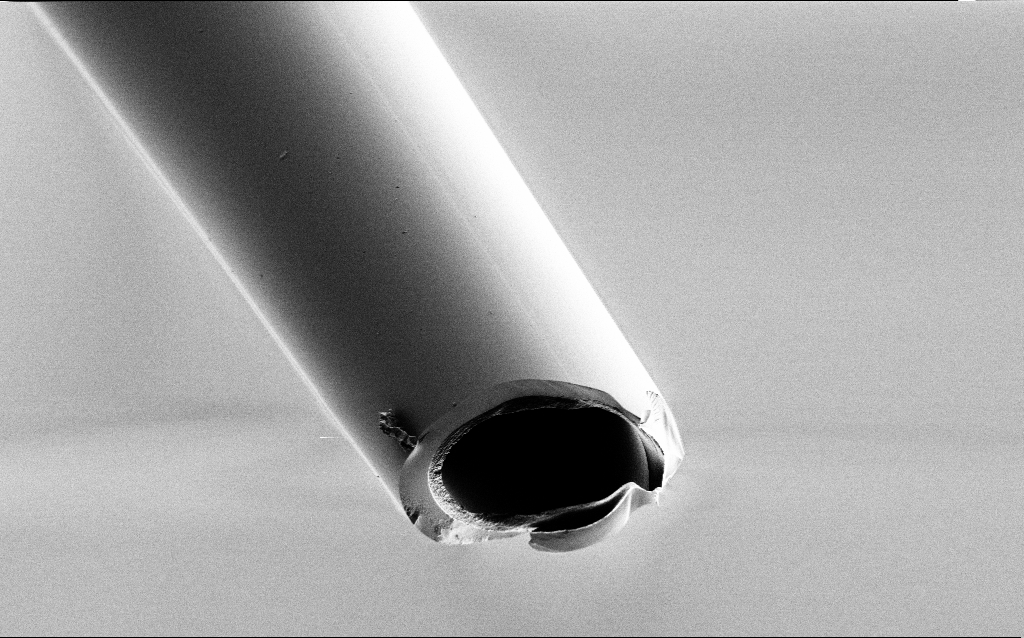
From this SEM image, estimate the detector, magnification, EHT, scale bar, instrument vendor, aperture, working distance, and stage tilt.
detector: SE2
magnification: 2.5 K X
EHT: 1 kV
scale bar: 10000 nm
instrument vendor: Zeiss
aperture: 30 µm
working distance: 7.5 mm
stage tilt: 45°